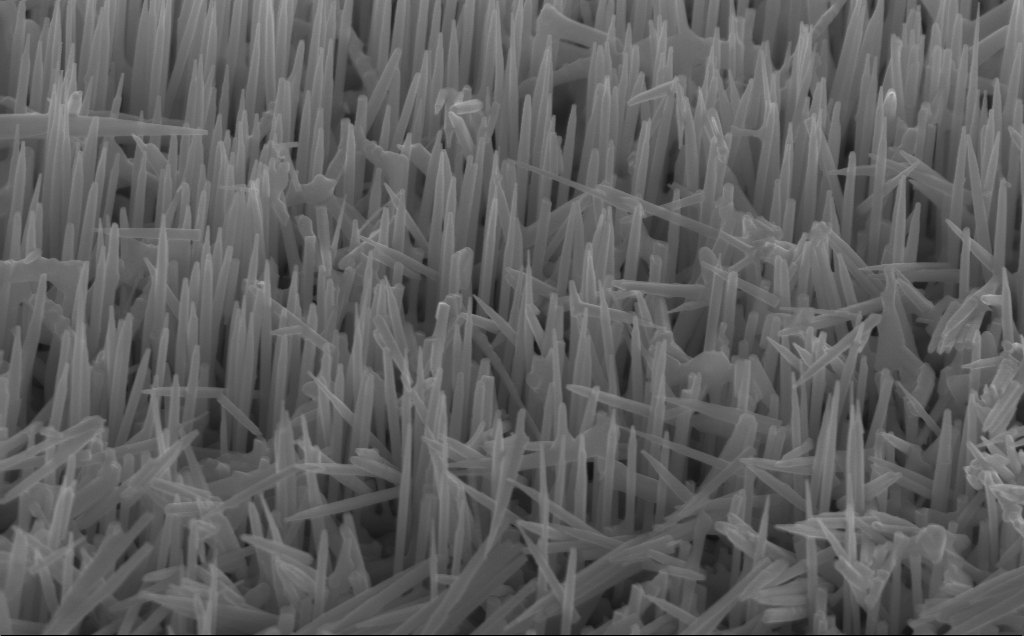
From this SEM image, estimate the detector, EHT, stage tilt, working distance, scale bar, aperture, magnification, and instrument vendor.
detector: InLens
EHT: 12 kV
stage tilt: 45°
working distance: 5 mm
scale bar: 1000 nm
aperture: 30 µm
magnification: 40 K X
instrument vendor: Zeiss